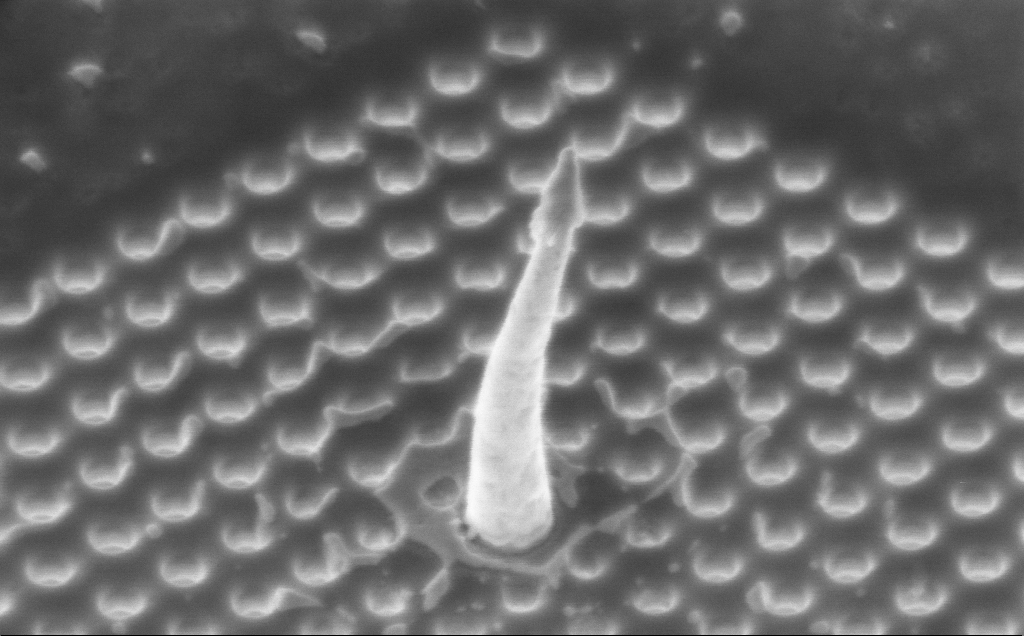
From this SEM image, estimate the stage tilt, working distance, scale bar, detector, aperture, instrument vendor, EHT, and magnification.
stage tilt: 34.3°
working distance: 6 mm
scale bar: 200 nm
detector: InLens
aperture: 30 µm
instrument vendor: Zeiss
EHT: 3 kV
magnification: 109.14 K X